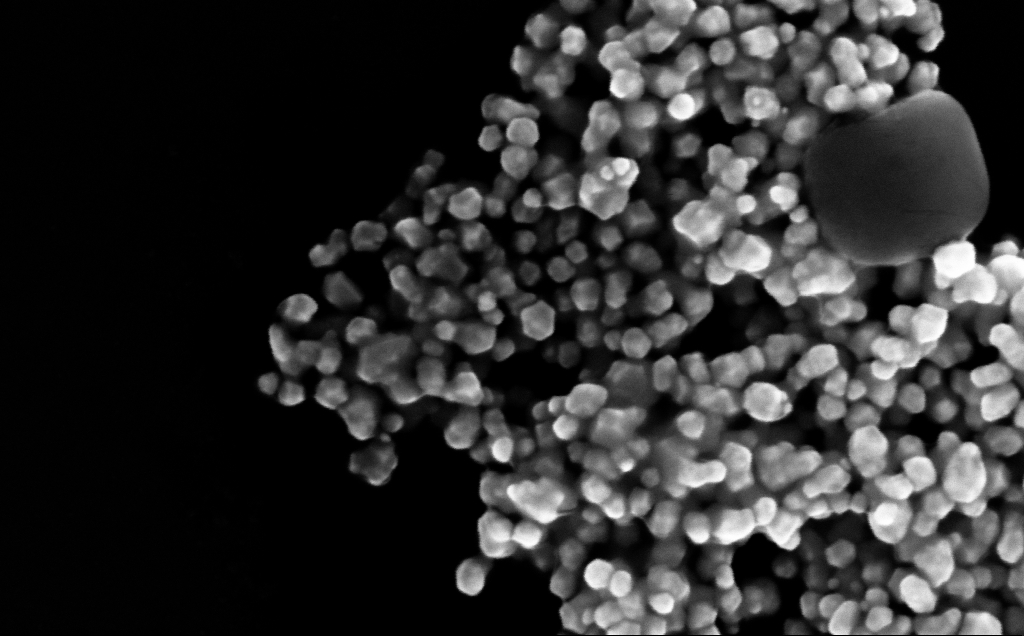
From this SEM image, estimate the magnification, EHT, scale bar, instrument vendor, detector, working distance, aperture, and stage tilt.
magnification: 288.95 K X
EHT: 10 kV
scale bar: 200 nm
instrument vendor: Zeiss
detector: InLens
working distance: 4 mm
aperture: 30 µm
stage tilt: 0°